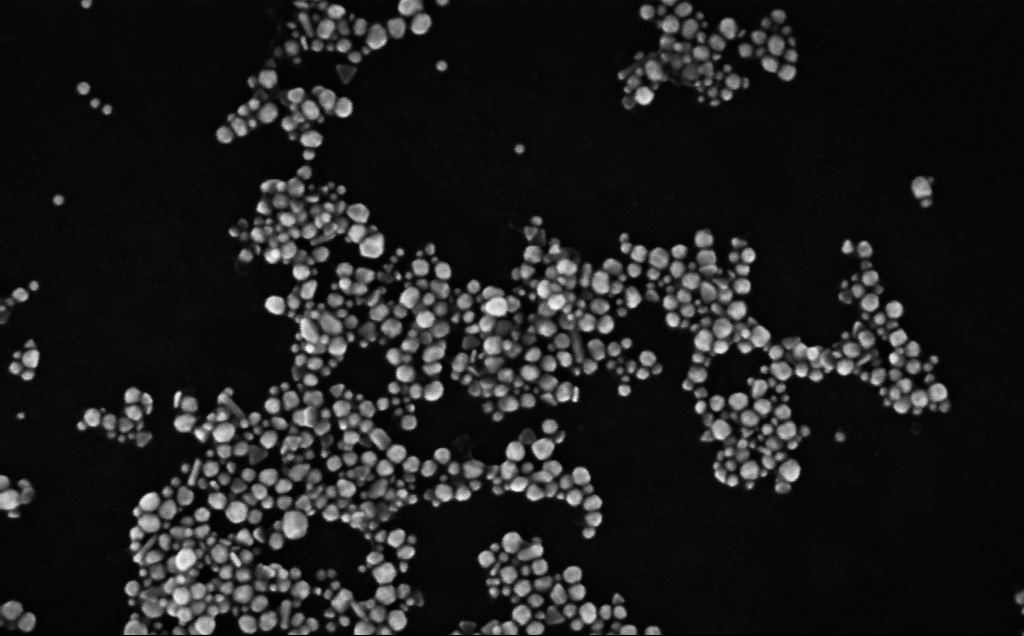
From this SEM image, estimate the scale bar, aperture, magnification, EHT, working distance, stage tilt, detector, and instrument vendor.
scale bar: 200 nm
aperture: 30 µm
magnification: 283.99 K X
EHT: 10 kV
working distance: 4 mm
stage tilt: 0°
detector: InLens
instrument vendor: Zeiss